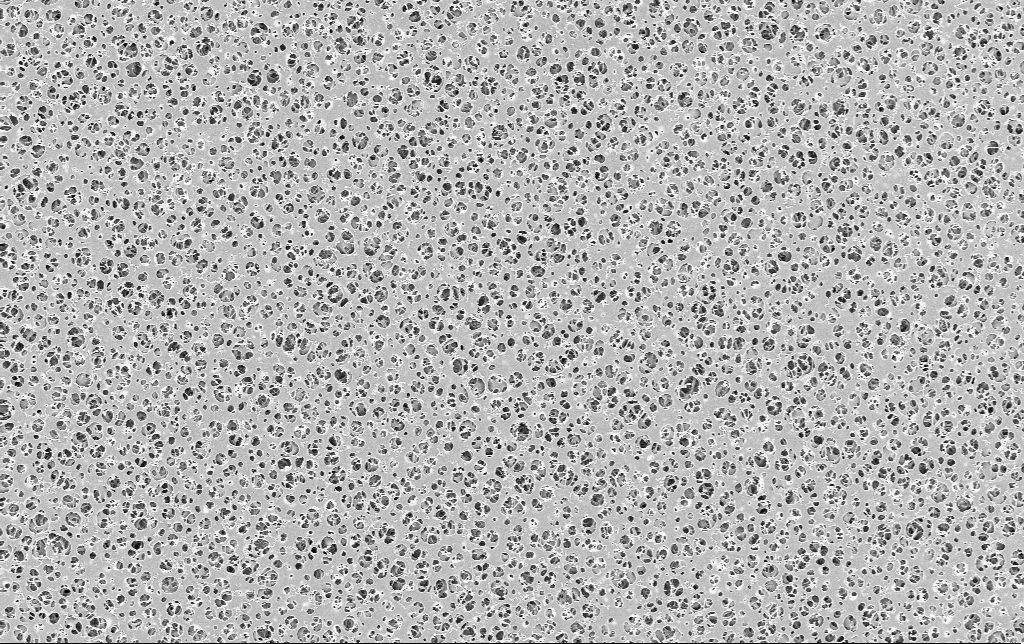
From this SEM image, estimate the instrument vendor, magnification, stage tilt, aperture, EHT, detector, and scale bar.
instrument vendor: Zeiss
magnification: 2.5 K X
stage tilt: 0°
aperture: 30 µm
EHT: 2 kV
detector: InLens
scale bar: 20000 nm